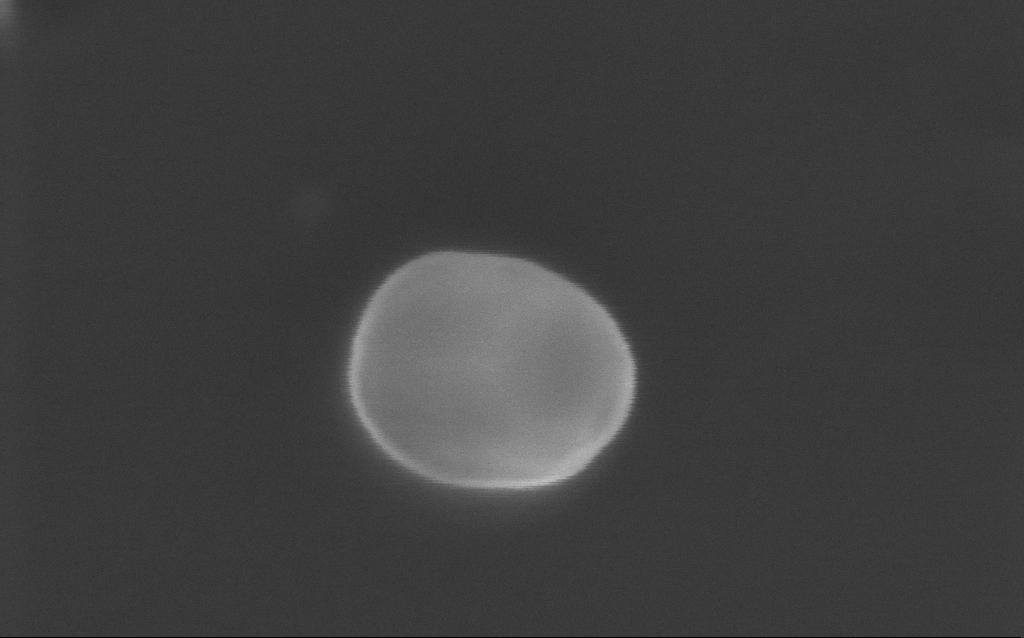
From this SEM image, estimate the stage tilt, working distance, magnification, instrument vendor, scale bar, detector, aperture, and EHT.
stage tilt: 0°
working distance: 3 mm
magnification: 473.93 K X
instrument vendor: Zeiss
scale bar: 100 nm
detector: InLens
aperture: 30 µm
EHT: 10 kV